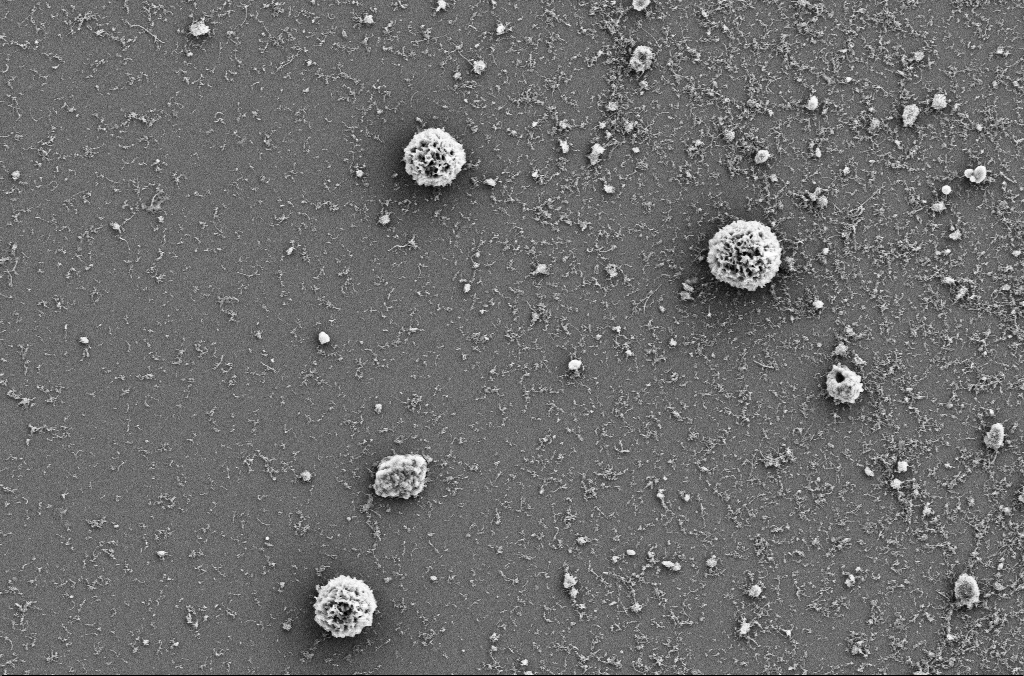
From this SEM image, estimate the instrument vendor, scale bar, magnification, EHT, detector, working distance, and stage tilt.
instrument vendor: Zeiss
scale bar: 10000 nm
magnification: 5 K X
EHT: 5 kV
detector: SE2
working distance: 4 mm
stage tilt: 0°